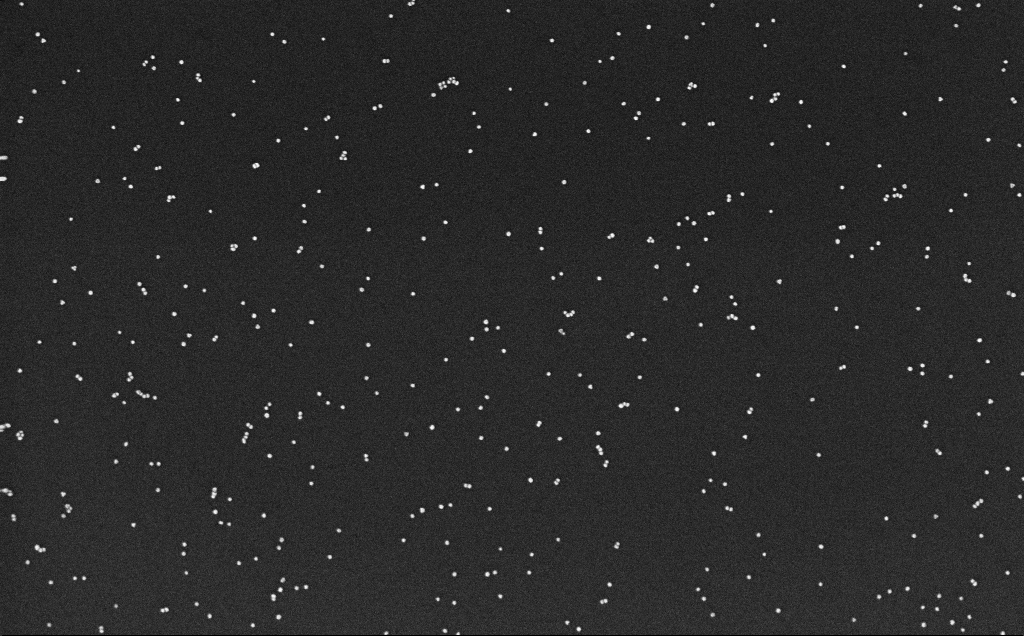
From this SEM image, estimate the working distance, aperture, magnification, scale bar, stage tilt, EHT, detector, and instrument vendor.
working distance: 3.2 mm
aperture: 30 µm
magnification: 100 K X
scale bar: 200 nm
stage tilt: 0°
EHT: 10 kV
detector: InLens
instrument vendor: Zeiss